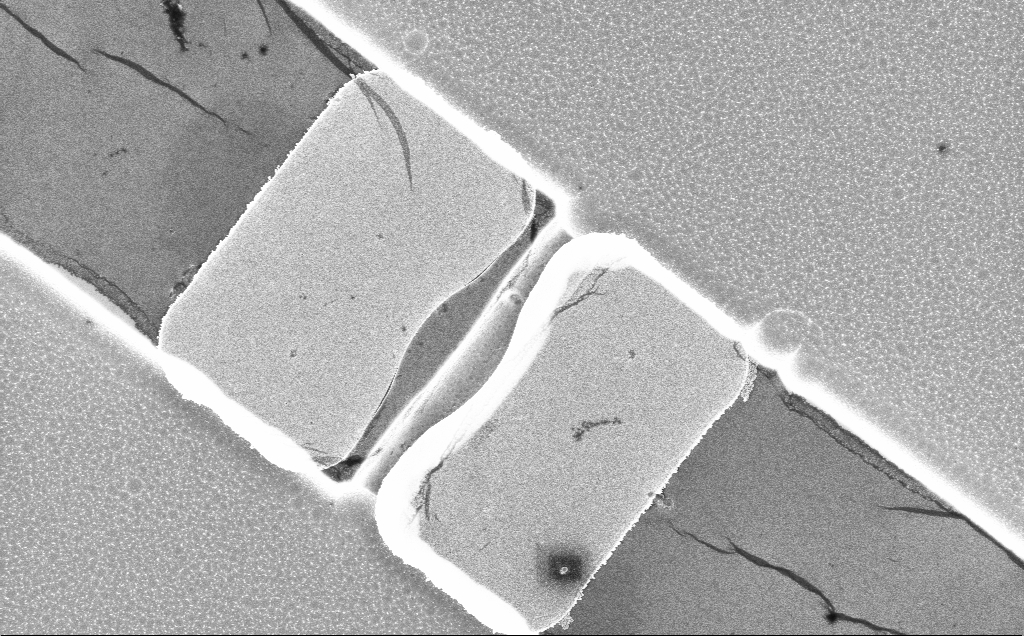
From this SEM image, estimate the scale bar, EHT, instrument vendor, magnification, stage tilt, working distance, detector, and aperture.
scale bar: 2000 nm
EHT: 5 kV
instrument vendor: Zeiss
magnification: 7.31 K X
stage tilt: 0°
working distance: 10 mm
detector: InLens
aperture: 30 µm